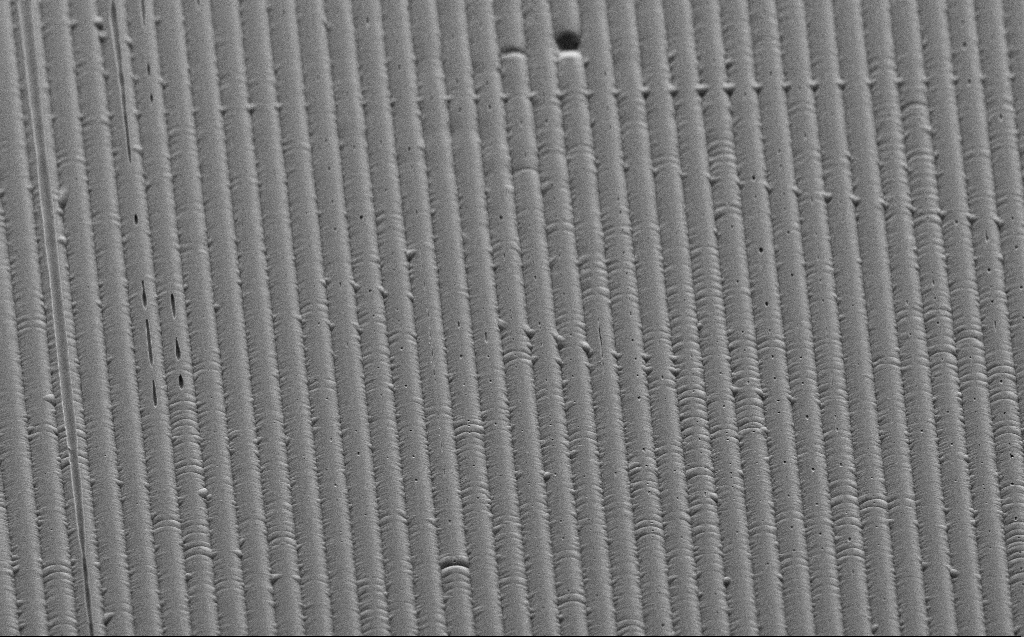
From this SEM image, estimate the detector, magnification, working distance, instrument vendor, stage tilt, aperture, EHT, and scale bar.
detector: SE2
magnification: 1.05 K X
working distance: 6 mm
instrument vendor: Zeiss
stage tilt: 45°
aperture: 30 µm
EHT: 3 kV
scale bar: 20000 nm